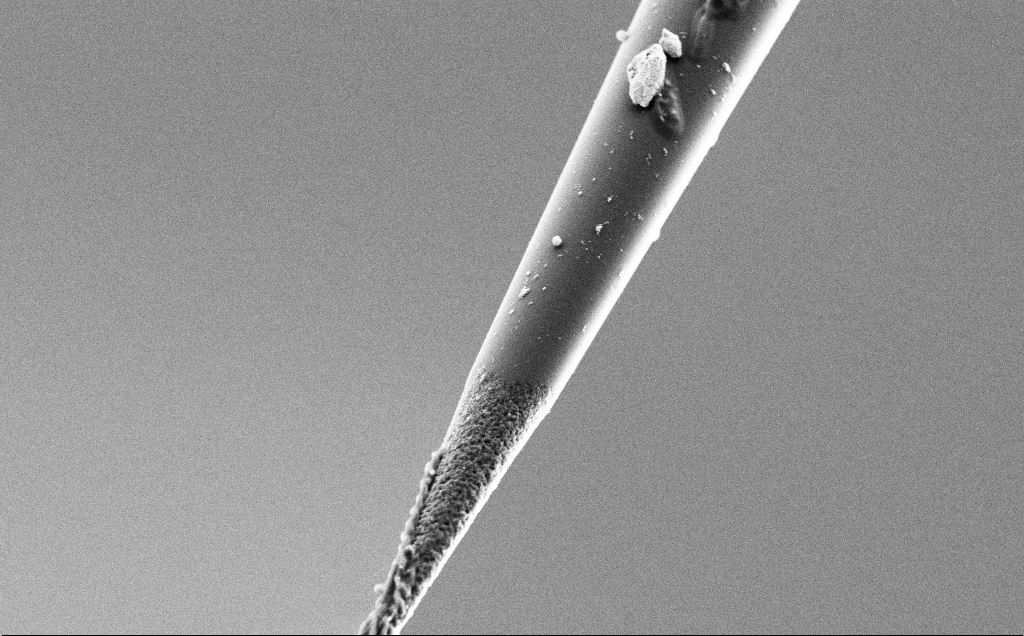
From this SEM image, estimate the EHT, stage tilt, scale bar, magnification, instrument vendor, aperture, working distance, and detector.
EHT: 3 kV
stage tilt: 45°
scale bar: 1000 nm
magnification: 15 K X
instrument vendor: Zeiss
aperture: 30 µm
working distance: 7.7 mm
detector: SE2